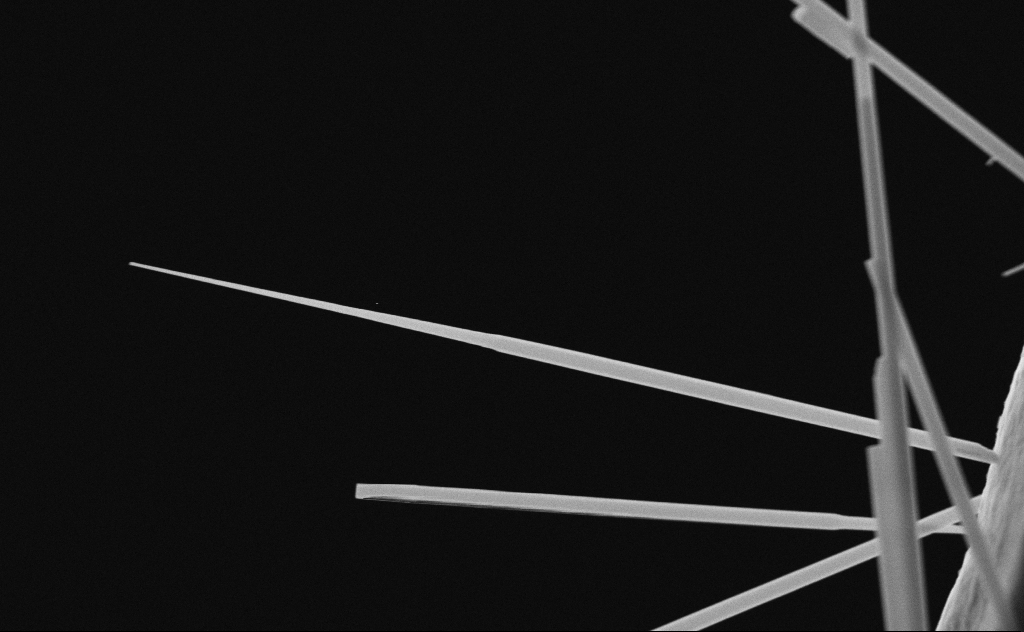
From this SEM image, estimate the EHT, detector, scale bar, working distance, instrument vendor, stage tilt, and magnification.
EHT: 20 kV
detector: SE2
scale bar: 1000 nm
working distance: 7 mm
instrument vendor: Zeiss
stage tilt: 0°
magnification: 43.84 K X